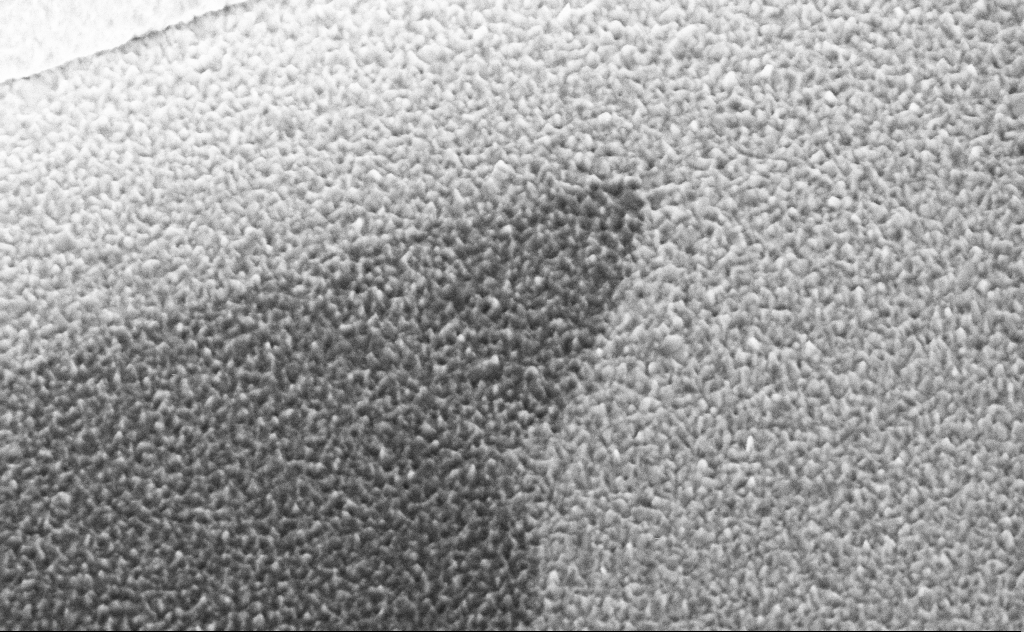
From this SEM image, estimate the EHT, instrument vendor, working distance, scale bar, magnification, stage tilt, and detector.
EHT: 5 kV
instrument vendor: Zeiss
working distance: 10 mm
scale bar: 1000 nm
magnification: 65.57 K X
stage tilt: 45°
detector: InLens